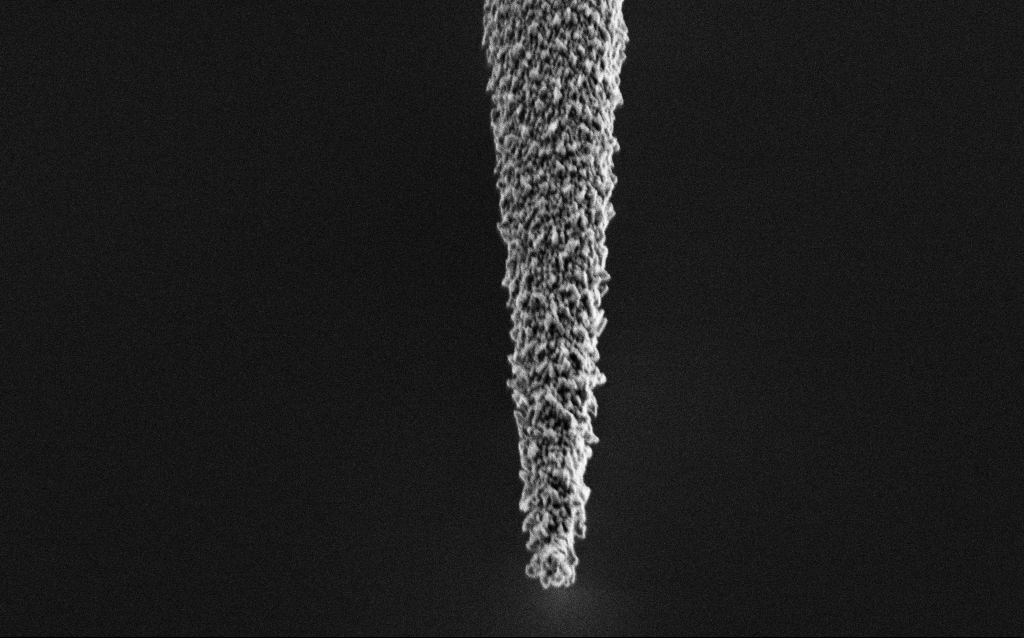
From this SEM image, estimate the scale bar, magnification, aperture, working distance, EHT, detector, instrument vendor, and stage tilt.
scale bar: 2000 nm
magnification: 25 K X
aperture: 30 µm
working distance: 6.8 mm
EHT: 2 kV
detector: SE2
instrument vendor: Zeiss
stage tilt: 44.9°